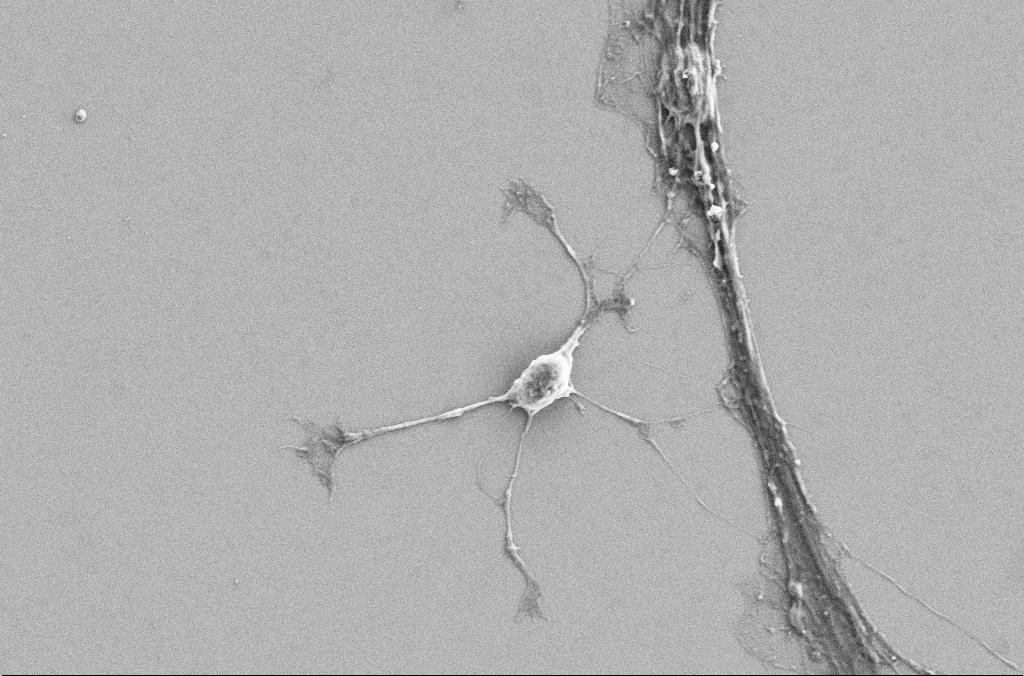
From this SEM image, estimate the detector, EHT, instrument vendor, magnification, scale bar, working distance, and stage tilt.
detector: SE2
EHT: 5 kV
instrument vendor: Zeiss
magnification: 4 K X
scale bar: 10000 nm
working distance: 3.2 mm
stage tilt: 0°